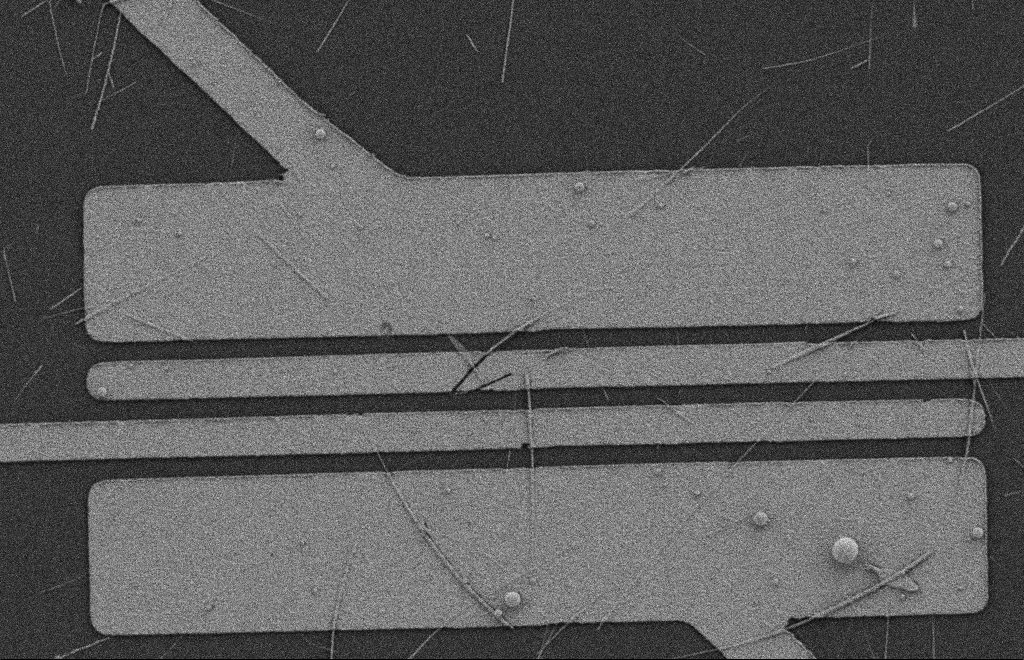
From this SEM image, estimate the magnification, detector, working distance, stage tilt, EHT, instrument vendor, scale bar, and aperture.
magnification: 5.39 K X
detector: SE2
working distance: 8 mm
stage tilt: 0°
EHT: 2 kV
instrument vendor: Zeiss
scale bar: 2000 nm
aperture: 20 µm